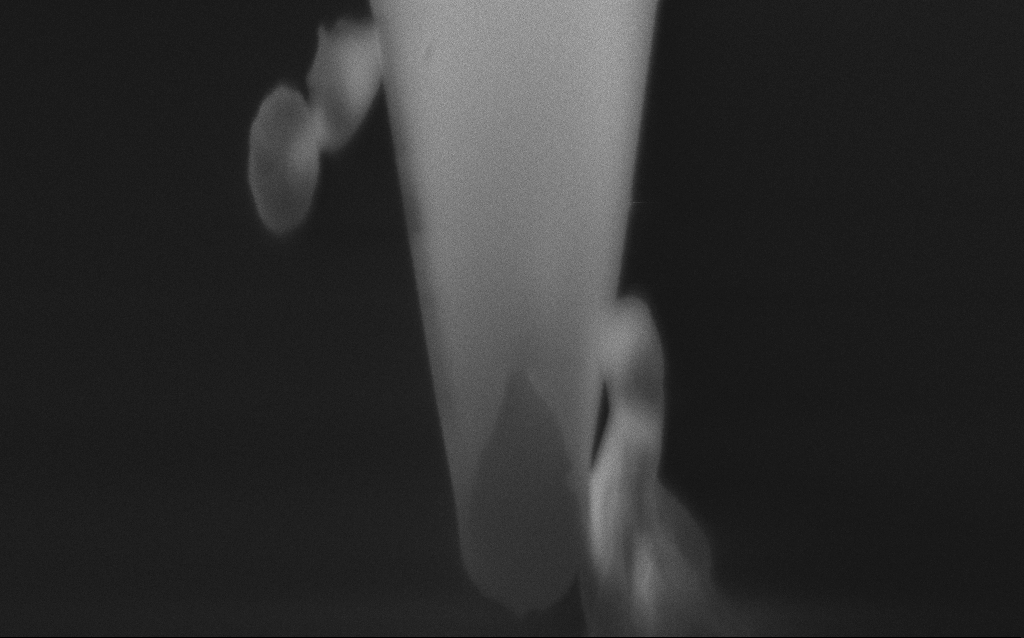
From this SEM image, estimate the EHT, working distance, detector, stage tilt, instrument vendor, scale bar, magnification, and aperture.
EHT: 2 kV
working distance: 4 mm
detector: InLens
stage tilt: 45°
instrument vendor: Zeiss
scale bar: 200 nm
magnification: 131.05 K X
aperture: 30 µm